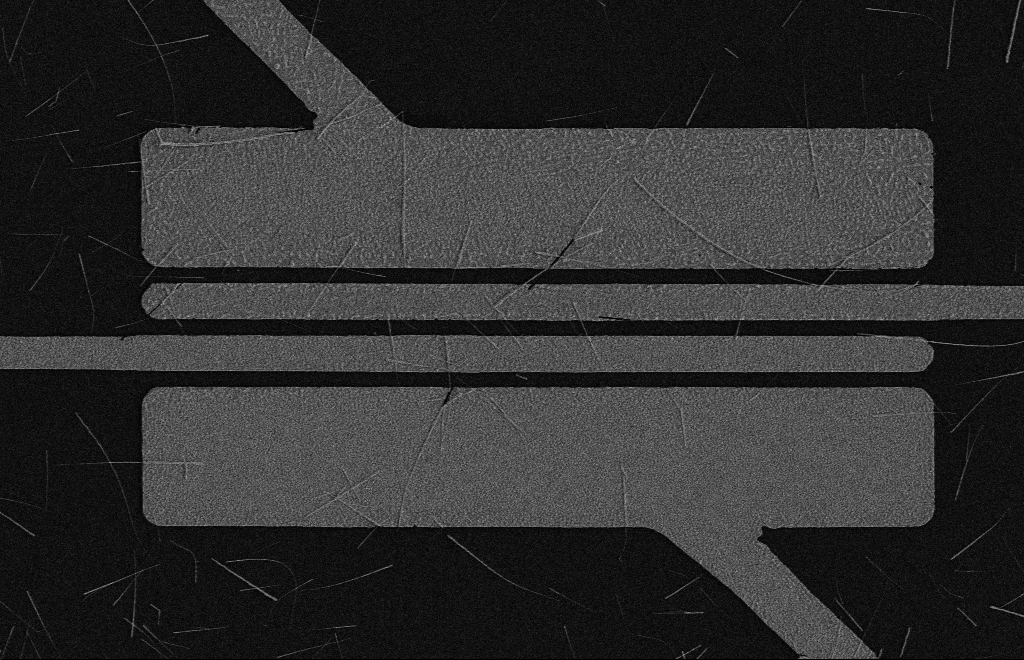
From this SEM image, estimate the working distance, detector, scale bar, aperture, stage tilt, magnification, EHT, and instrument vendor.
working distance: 16 mm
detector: SE2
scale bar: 2000 nm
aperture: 10 µm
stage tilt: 0°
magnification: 4.79 K X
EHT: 5 kV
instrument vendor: Zeiss